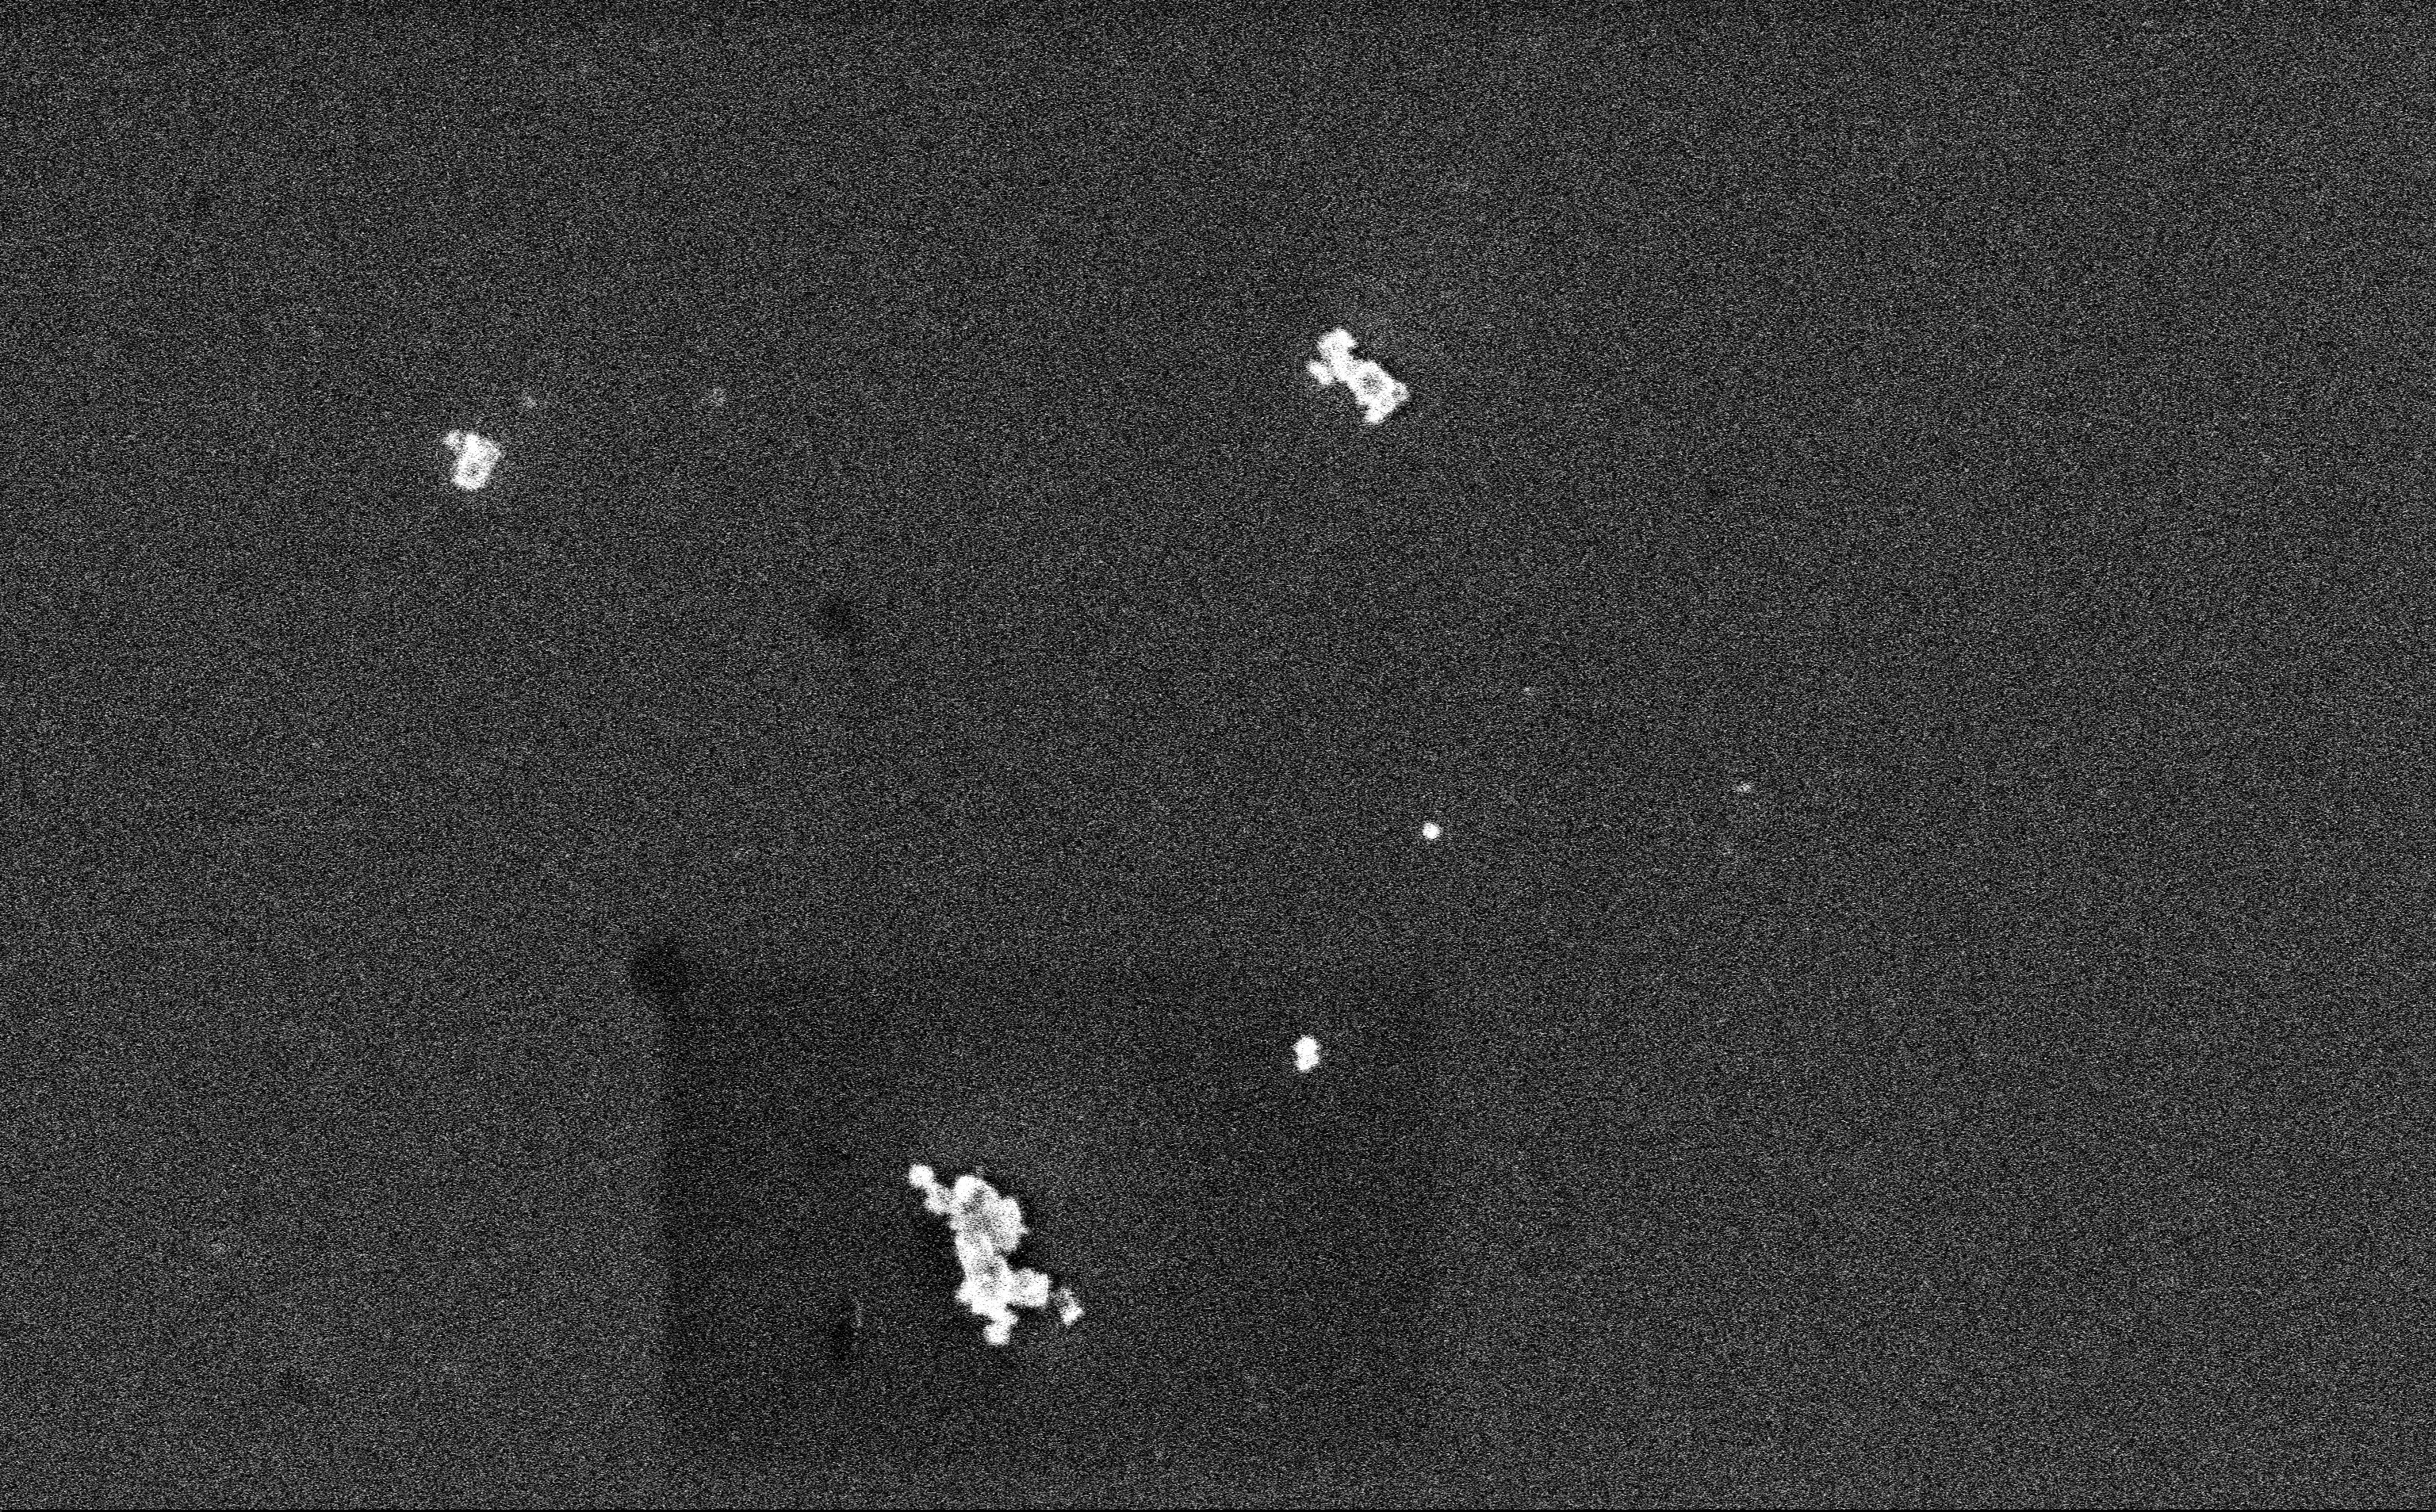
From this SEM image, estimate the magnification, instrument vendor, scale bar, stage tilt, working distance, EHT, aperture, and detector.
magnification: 27.35 K X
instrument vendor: Zeiss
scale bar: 1000 nm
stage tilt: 0°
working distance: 3 mm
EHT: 3 kV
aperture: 30 µm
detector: InLens